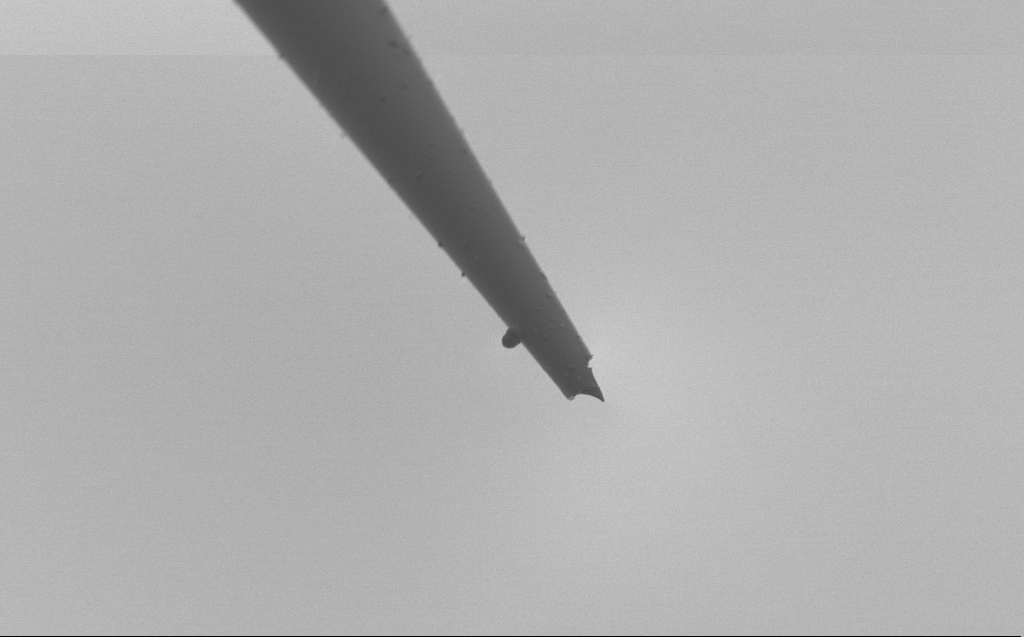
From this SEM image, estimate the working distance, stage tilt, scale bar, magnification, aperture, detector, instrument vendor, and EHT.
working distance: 4 mm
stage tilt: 45°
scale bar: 10000 nm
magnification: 5 K X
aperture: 30 µm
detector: SE2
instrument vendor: Zeiss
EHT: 2 kV